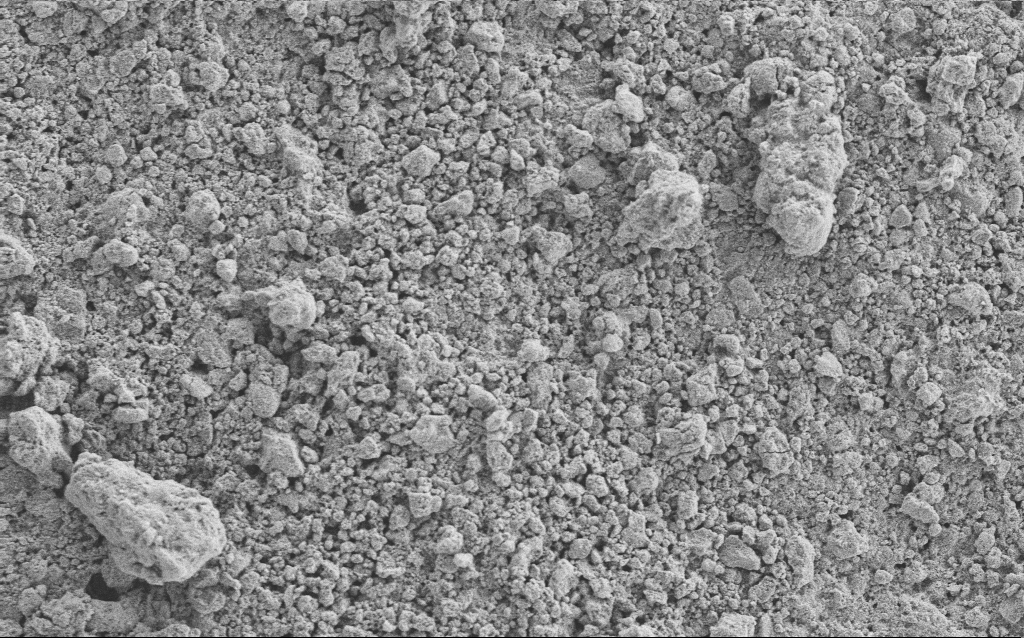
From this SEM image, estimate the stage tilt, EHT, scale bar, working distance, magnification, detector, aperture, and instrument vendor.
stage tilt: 0°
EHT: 5 kV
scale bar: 10000 nm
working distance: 4.7 mm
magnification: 1.23 K X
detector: SE2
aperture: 30 µm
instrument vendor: Zeiss